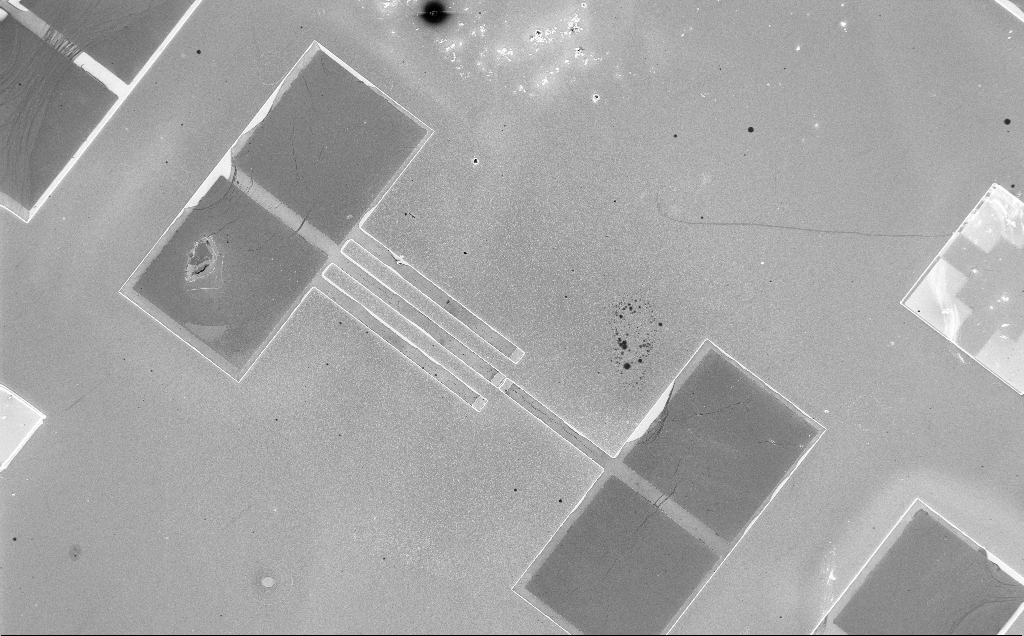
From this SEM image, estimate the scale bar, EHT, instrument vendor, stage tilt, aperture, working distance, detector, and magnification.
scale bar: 100000 nm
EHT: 5 kV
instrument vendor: Zeiss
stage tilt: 0°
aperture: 30 µm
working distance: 10 mm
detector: InLens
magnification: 0.281 K X